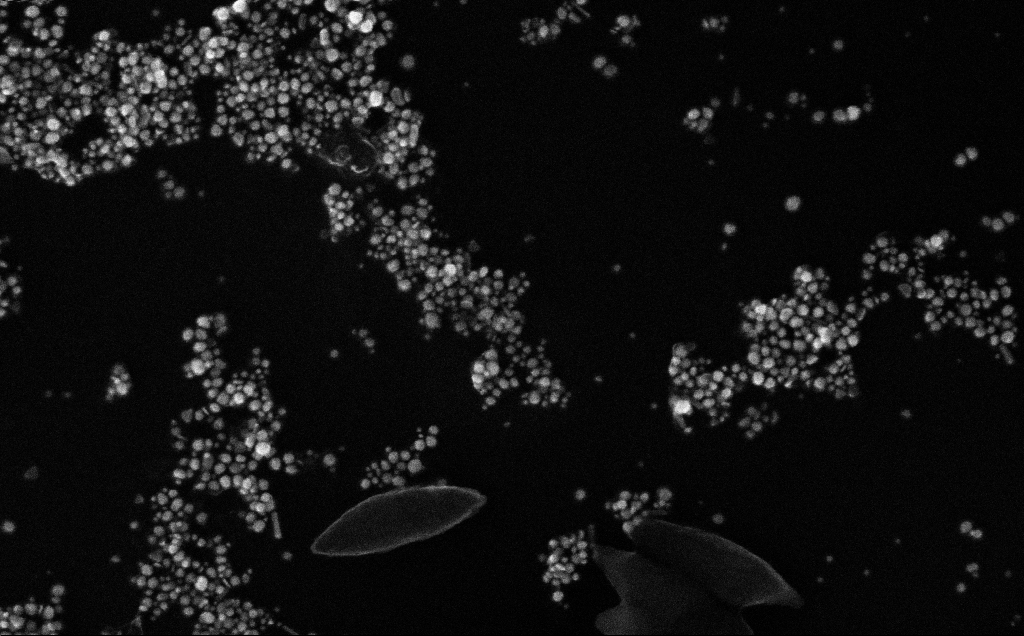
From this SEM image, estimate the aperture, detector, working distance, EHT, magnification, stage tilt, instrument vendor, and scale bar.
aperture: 30 µm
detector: InLens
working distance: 4 mm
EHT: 10 kV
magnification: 177.26 K X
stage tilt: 0°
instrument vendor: Zeiss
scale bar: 100 nm